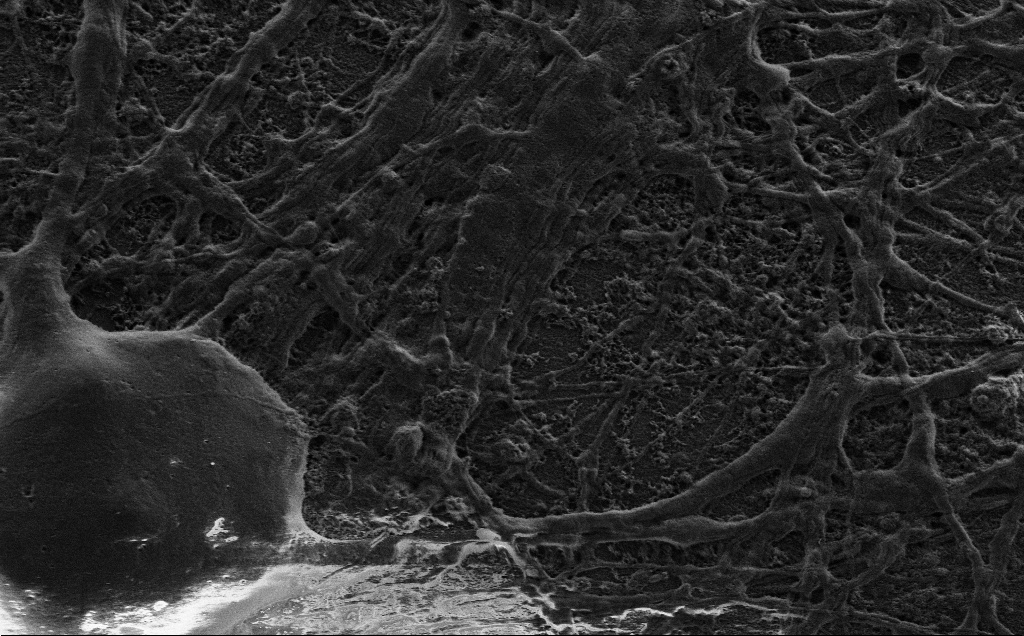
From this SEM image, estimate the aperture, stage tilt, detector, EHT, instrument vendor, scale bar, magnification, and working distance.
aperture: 30 µm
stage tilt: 0°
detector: SE2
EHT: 1.5 kV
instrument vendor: Zeiss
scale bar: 2000 nm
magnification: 11.52 K X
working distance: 4 mm